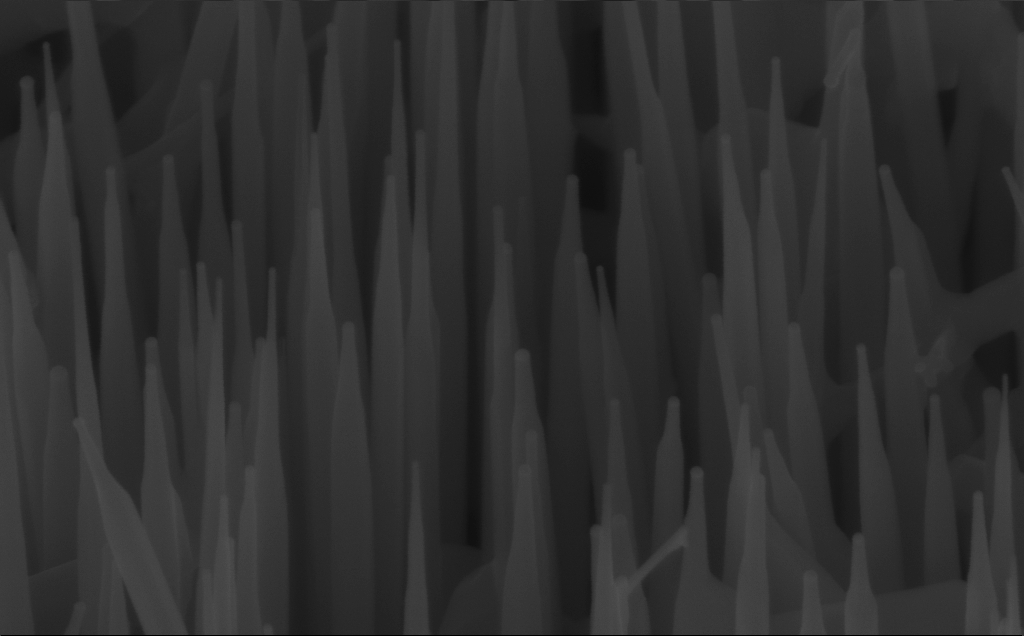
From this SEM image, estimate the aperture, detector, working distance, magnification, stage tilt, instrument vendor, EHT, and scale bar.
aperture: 30 µm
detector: InLens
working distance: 7 mm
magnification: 150 K X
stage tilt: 45°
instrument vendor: Zeiss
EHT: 10 kV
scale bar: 100 nm